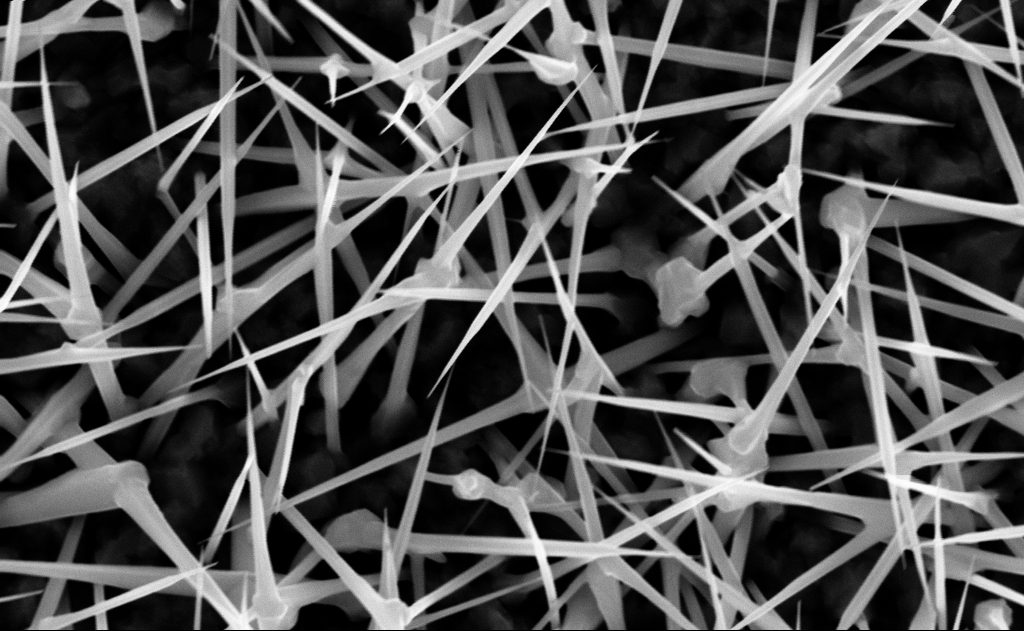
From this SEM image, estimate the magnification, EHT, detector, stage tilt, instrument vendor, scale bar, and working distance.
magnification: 60 K X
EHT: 10 kV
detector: InLens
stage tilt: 0°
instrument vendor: Zeiss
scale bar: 1000 nm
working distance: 9 mm